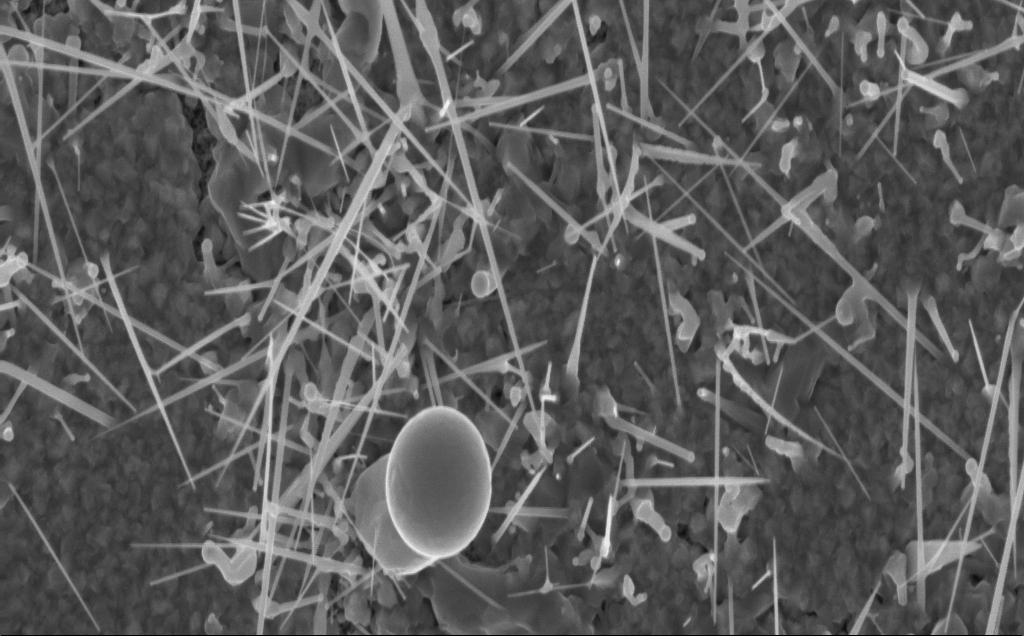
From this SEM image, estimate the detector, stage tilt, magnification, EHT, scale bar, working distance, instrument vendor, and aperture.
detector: InLens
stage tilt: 0°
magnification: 20 K X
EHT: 10 kV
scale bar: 2000 nm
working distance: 4 mm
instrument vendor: Zeiss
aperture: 30 µm